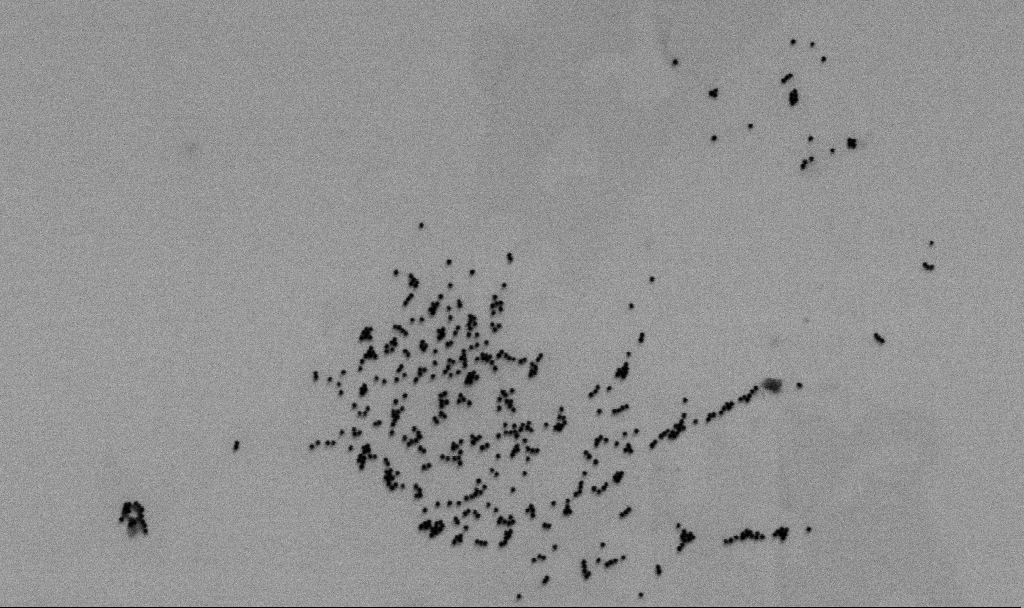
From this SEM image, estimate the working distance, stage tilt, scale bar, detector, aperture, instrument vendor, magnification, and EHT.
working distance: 6.5 mm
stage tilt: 0°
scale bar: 200 nm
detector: SE2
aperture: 30 µm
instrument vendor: Zeiss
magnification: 75.26 K X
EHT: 2 kV